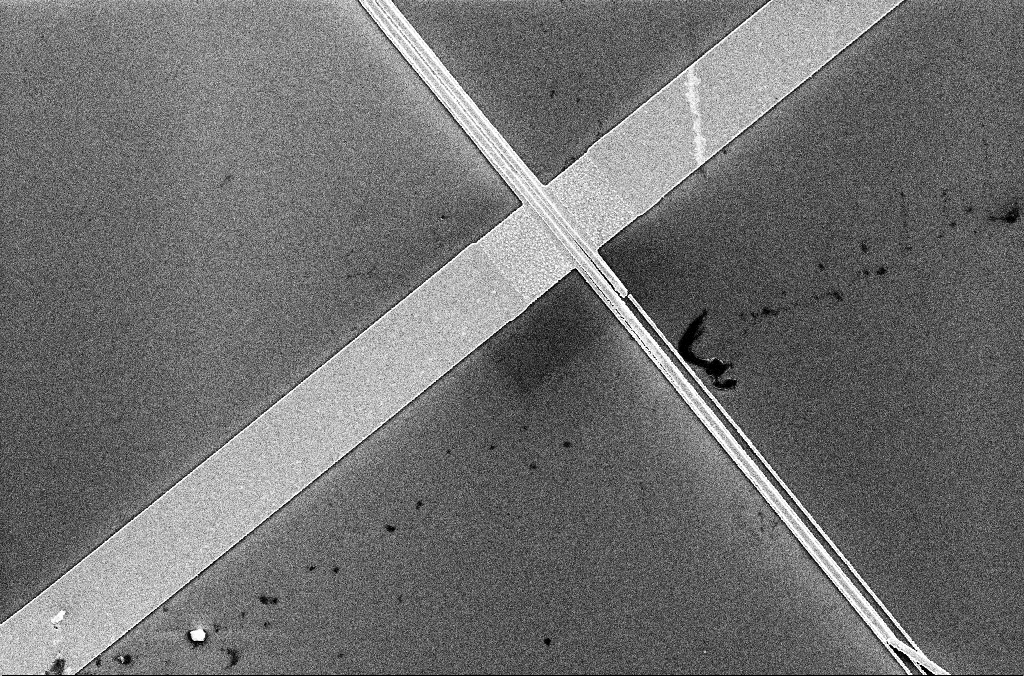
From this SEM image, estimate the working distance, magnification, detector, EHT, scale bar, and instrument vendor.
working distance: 3.4 mm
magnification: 6.14 K X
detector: InLens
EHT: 5 kV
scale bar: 10000 nm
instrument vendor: Zeiss